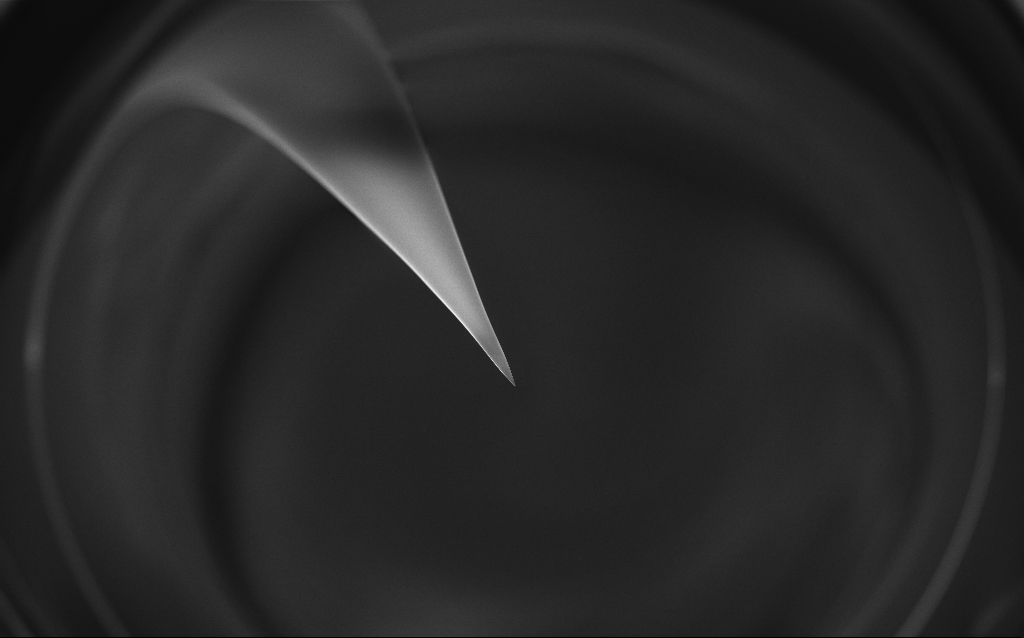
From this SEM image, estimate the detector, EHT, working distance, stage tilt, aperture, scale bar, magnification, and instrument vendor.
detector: InLens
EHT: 1 kV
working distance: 7 mm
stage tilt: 45°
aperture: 30 µm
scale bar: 200000 nm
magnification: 0.1 K X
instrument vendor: Zeiss